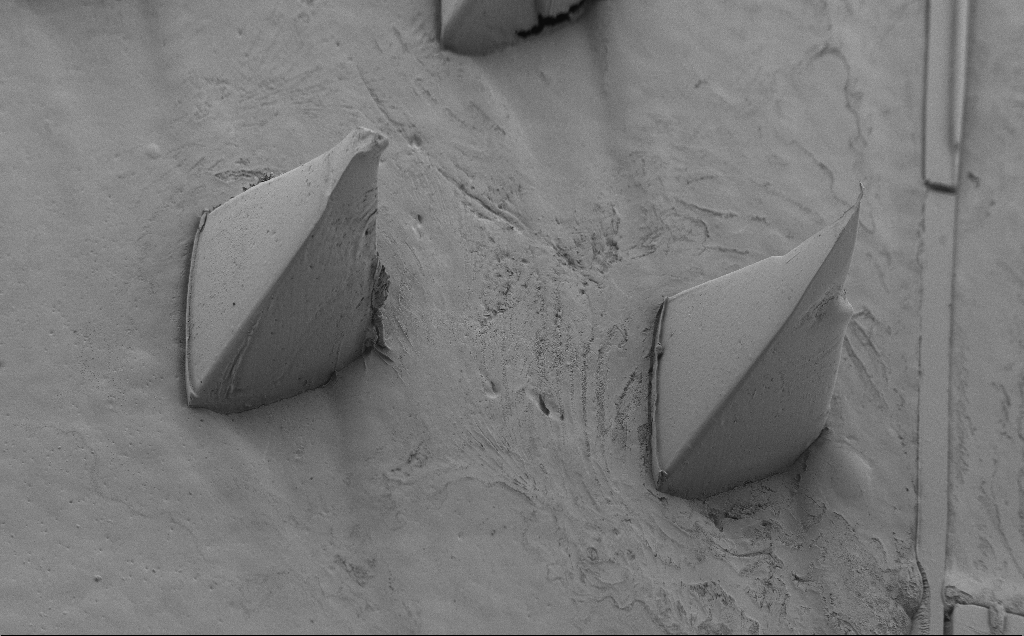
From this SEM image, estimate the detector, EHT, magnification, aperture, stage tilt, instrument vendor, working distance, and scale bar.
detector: SE2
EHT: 5 kV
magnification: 0.12 K X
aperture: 30 µm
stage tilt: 40°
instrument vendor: Zeiss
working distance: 8 mm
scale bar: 200000 nm